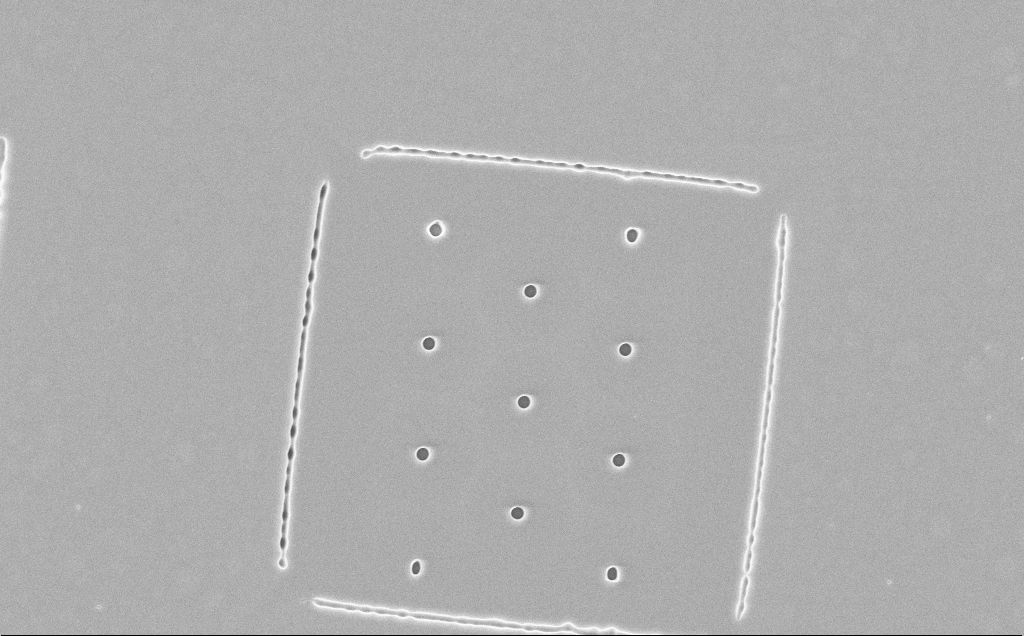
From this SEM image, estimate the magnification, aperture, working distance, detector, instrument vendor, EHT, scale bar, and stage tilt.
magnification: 2.03 K X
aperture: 30 µm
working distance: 16 mm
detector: InLens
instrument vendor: Zeiss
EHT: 10 kV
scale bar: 20000 nm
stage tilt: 0°